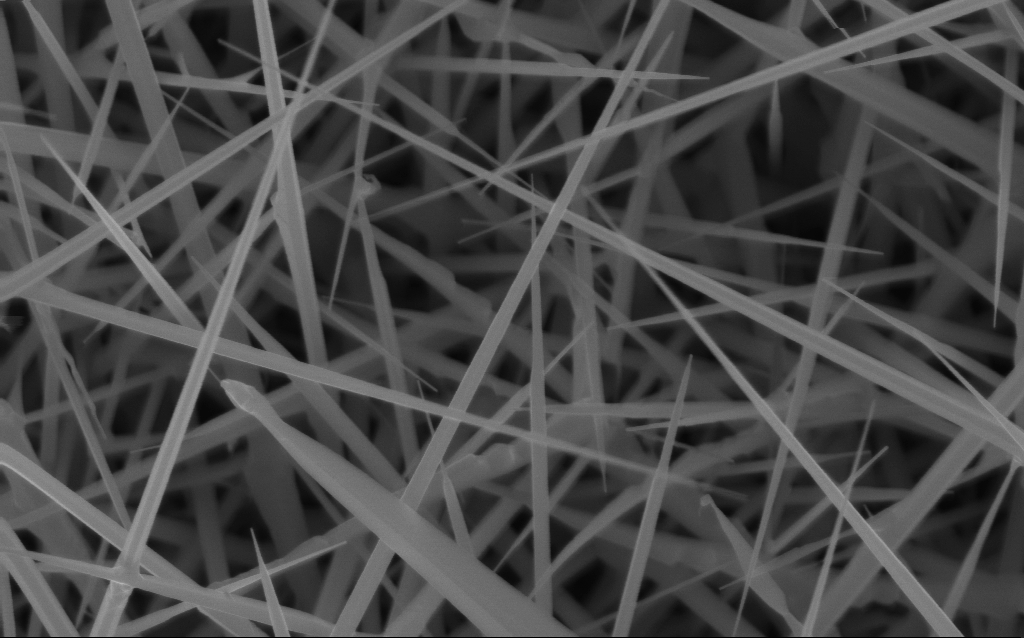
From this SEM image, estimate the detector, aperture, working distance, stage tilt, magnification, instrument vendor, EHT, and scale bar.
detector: InLens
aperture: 30 µm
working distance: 4 mm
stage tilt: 0°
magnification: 40 K X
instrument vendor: Zeiss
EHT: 10 kV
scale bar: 1000 nm